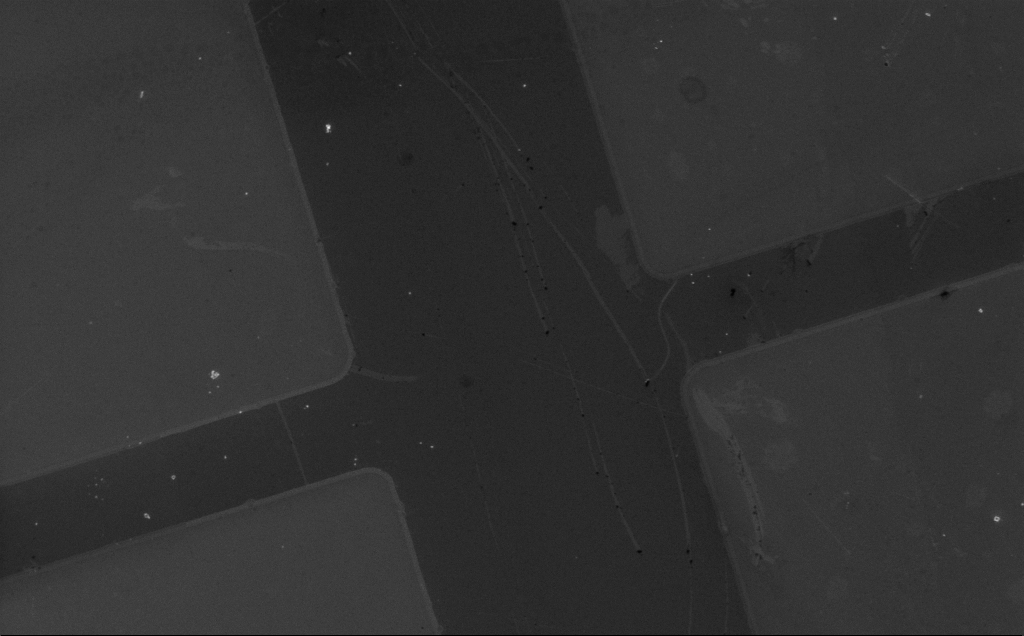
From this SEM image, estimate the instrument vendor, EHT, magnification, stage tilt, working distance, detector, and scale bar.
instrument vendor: Zeiss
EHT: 5 kV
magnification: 1.95 K X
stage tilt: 0°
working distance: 6 mm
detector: InLens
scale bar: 20000 nm